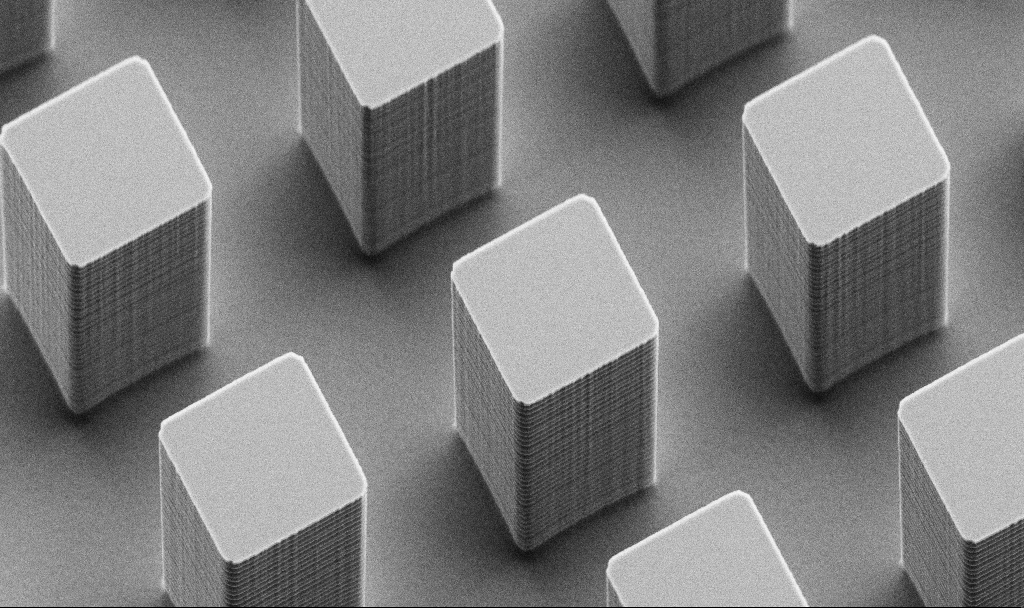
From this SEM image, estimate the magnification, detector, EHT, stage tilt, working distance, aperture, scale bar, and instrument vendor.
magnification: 6.09 K X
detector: SE2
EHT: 5 kV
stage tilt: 45°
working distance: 6.3 mm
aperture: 30 µm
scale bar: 10000 nm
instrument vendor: Zeiss